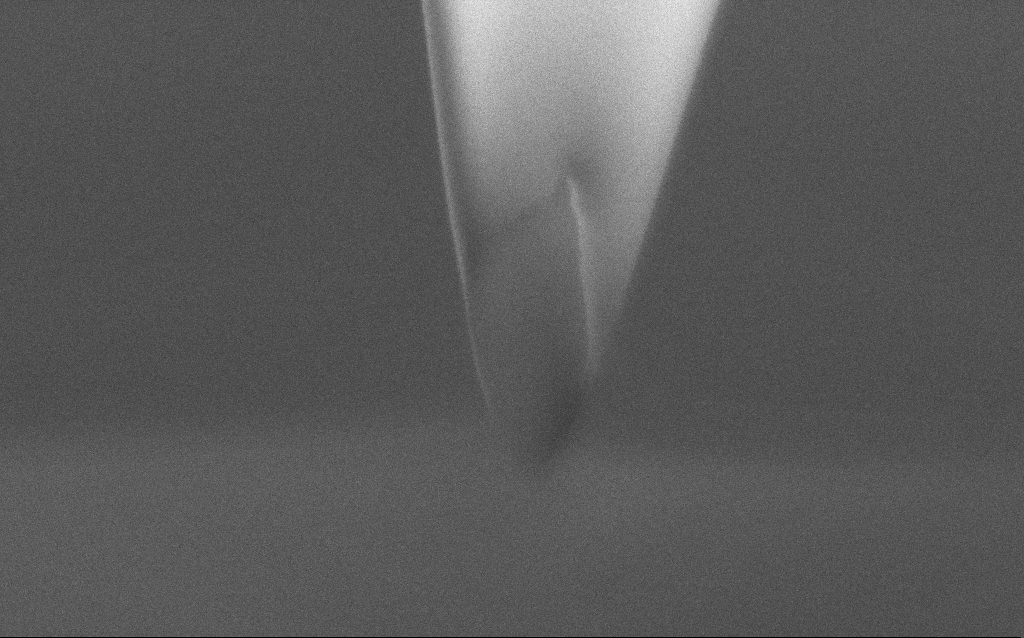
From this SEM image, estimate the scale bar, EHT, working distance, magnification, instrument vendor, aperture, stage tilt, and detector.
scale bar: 200 nm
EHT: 2 kV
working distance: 5 mm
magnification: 185.29 K X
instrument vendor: Zeiss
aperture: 30 µm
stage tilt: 45°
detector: InLens